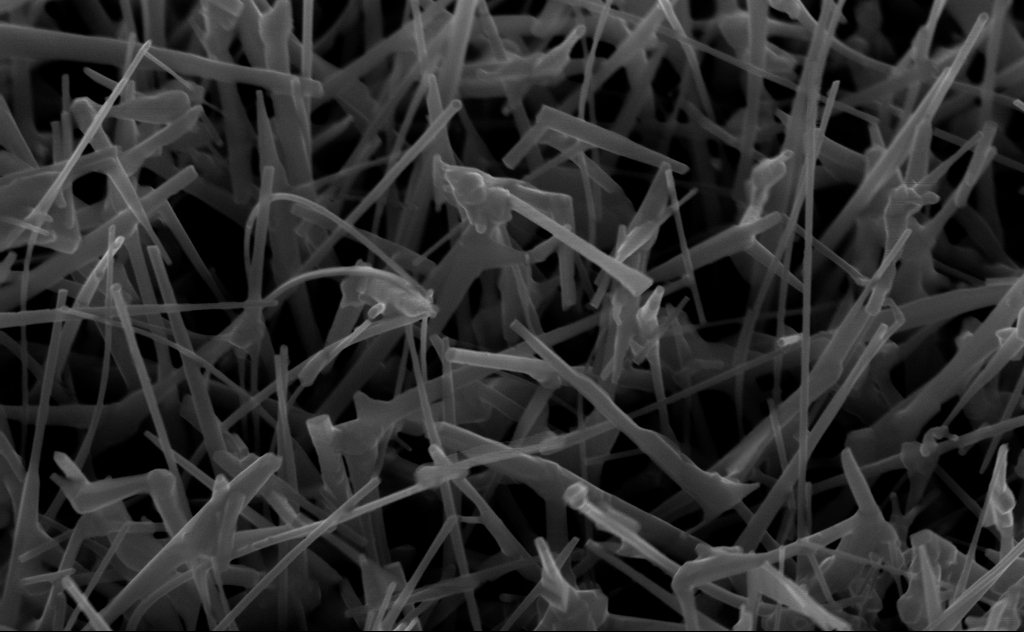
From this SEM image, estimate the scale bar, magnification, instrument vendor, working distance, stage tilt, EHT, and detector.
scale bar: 200 nm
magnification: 80 K X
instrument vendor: Zeiss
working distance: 5 mm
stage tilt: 45°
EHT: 10 kV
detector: InLens